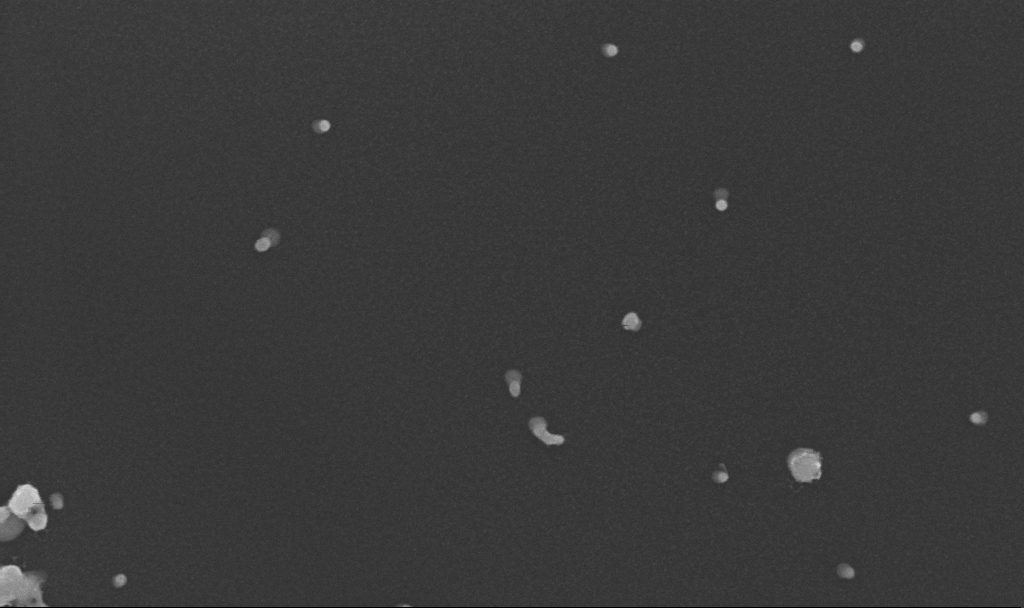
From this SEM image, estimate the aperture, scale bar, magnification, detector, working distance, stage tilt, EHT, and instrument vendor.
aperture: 30 µm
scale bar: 100 nm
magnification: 200 K X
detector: InLens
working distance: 3.3 mm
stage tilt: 0°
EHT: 10 kV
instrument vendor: Zeiss